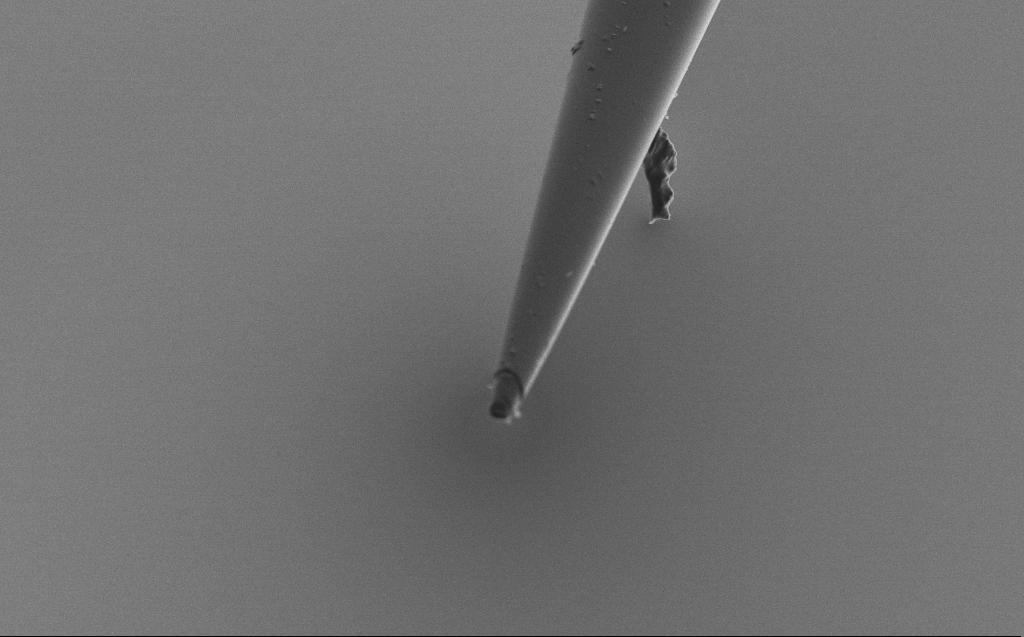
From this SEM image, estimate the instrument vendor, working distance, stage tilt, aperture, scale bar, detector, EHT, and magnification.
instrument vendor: Zeiss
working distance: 5 mm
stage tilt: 45°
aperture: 30 µm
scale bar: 2000 nm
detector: SE2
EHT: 2 kV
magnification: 10 K X